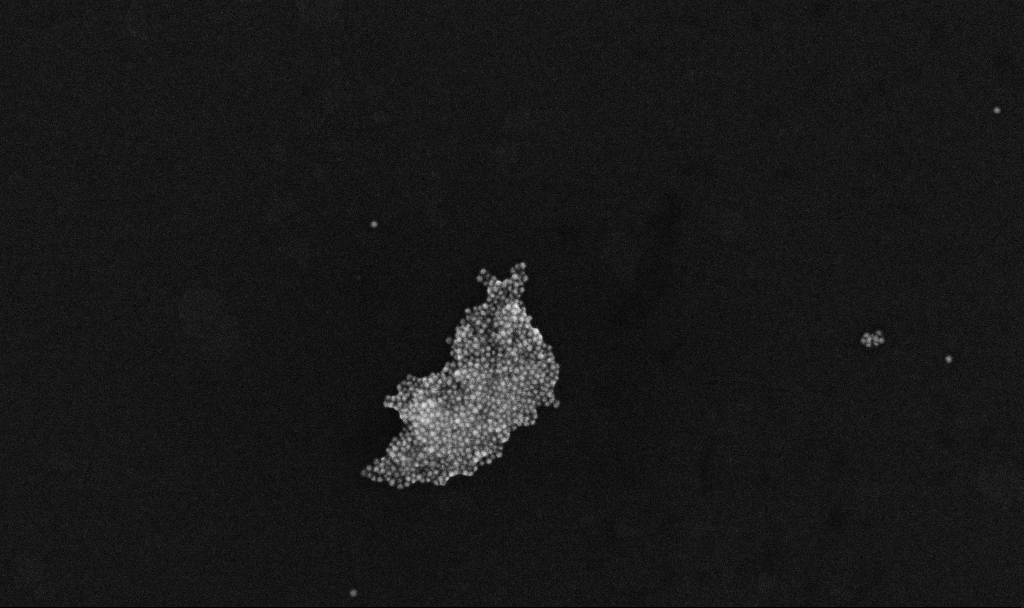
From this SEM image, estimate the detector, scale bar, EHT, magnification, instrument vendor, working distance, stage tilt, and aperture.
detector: InLens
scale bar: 200 nm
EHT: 10 kV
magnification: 100 K X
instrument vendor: Zeiss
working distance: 3.3 mm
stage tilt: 0°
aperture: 30 µm